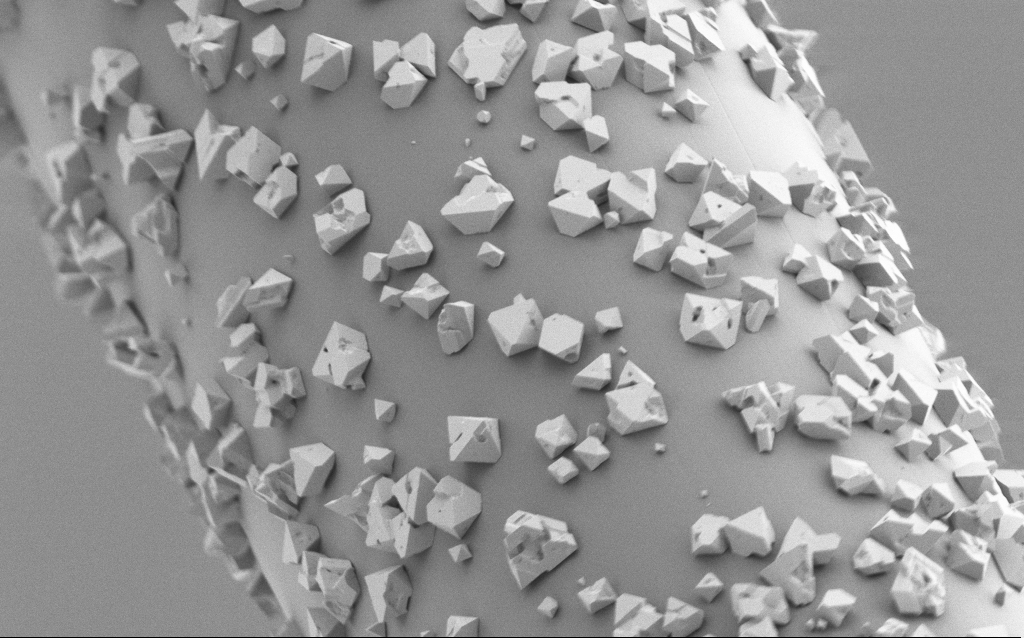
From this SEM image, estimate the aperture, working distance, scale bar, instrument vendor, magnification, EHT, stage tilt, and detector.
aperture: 30 µm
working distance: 7.6 mm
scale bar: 2000 nm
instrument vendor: Zeiss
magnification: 10 K X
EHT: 1.5 kV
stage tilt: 45°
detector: SE2